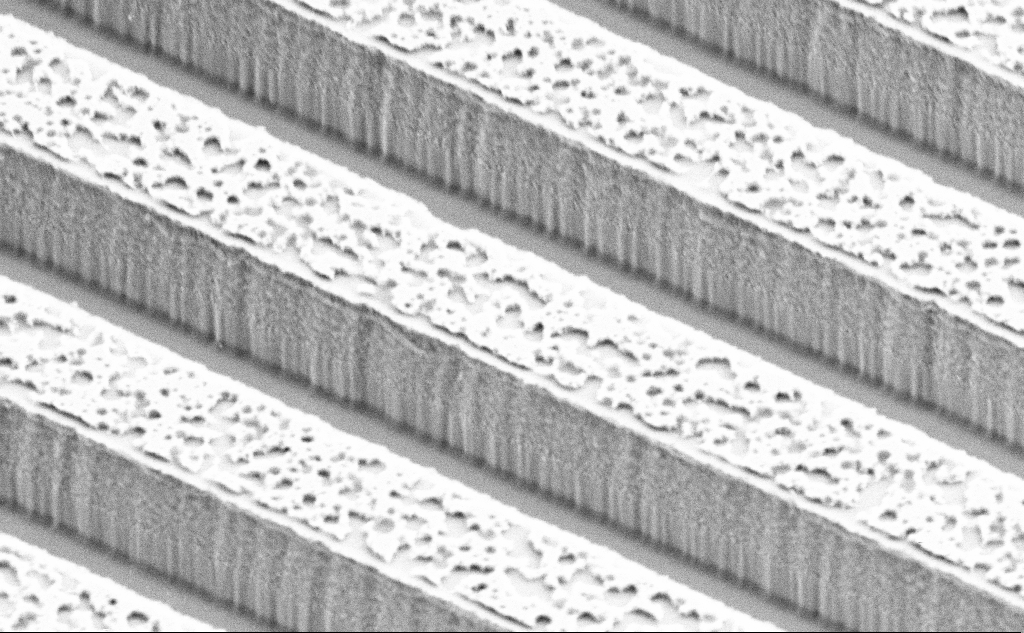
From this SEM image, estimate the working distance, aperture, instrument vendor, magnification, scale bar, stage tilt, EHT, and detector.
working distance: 12.8 mm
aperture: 30 µm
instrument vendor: Zeiss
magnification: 28.68 K X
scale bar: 1000 nm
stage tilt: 45°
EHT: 3 kV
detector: SE2